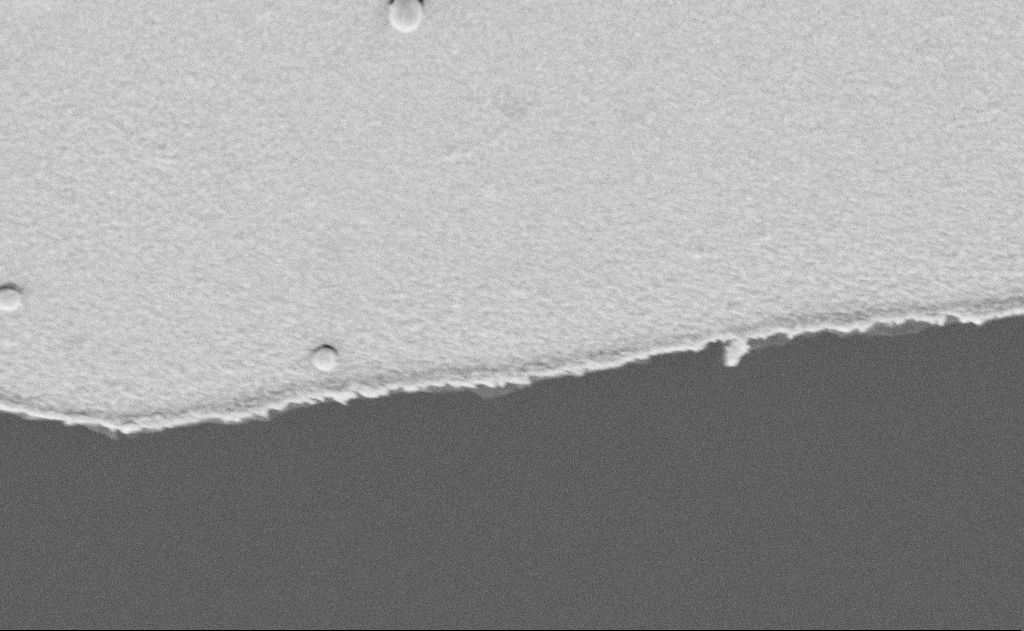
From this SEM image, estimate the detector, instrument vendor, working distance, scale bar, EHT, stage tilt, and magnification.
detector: SE2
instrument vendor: Zeiss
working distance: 8 mm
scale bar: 1000 nm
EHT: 5 kV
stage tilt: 39.8°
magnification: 50.88 K X